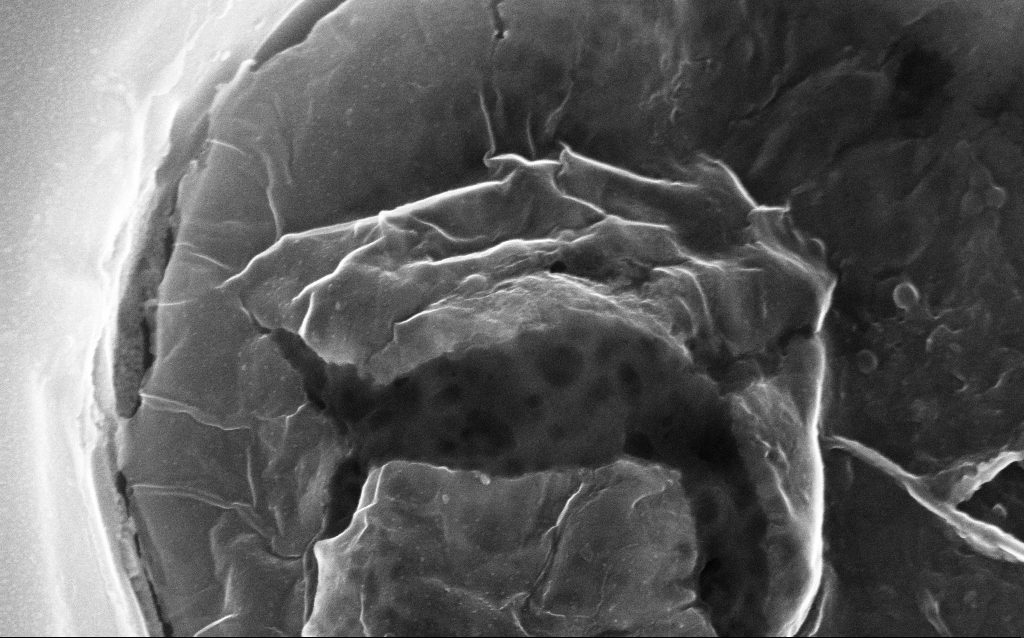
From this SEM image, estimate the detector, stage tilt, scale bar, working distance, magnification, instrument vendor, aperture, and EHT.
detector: InLens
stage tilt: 21.2°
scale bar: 200 nm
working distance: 5.7 mm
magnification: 97.96 K X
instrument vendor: Zeiss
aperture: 30 µm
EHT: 20 kV